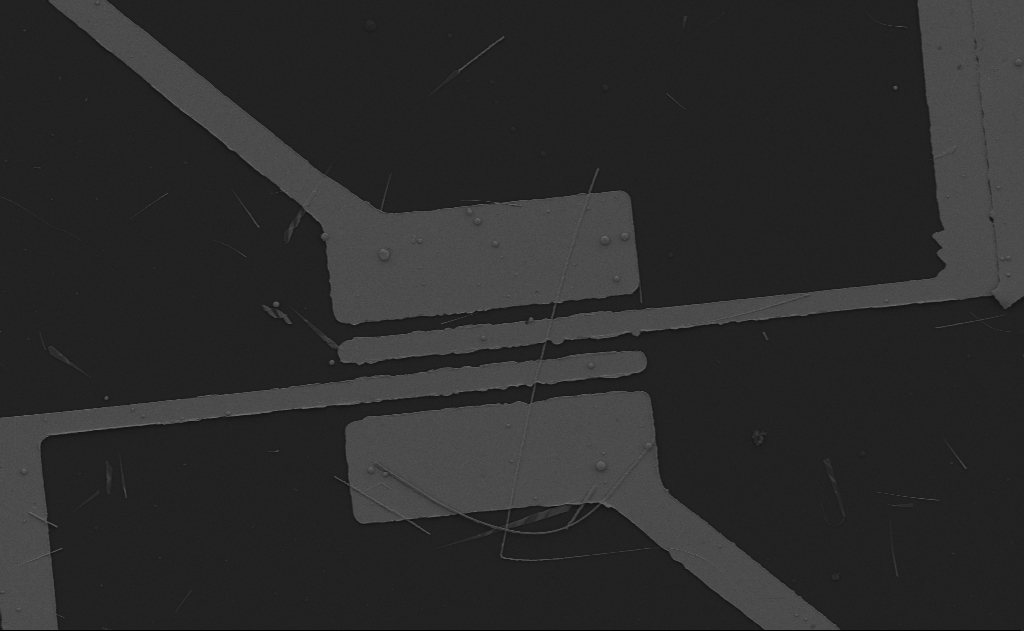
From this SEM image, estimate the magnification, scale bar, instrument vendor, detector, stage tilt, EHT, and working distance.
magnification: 3.73 K X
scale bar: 10000 nm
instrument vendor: Zeiss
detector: SE2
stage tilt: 0°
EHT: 5 kV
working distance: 13 mm